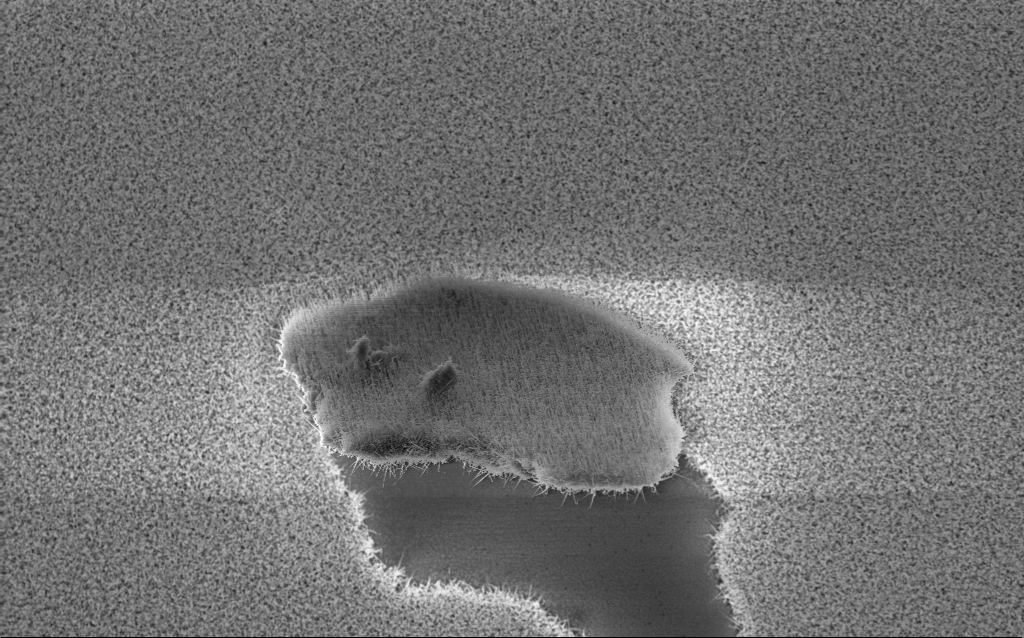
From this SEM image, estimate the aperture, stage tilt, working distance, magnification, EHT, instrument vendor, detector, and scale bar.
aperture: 30 µm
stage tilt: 0°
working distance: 7.6 mm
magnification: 5.67 K X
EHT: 10 kV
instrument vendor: Zeiss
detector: InLens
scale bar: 10000 nm